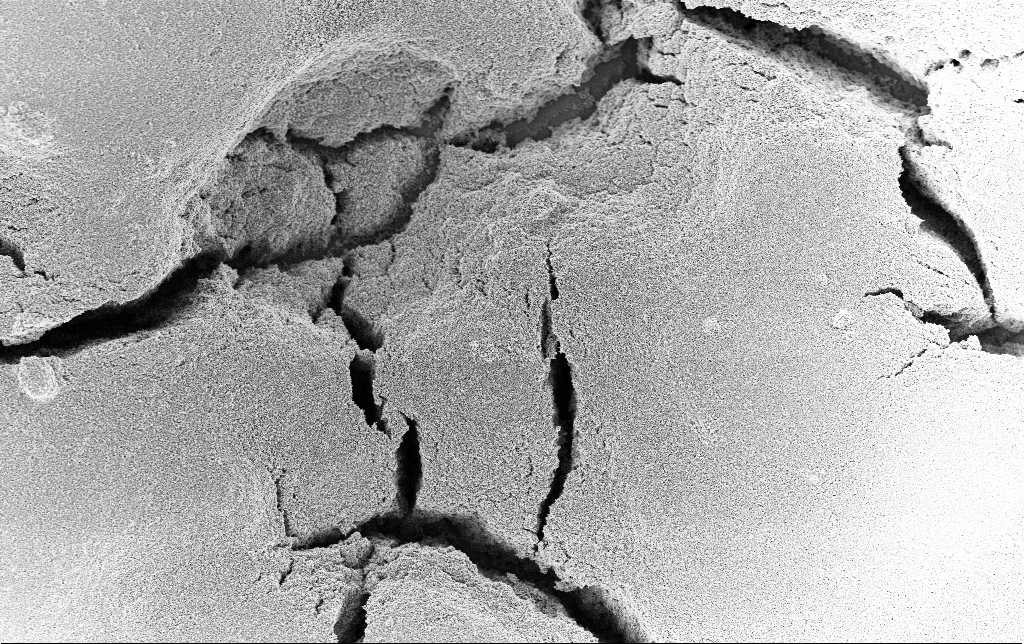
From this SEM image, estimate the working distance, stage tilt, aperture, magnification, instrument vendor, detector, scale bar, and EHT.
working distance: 2.4 mm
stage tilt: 0°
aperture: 30 µm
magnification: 3 K X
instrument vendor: Zeiss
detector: InLens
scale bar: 20000 nm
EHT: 3 kV